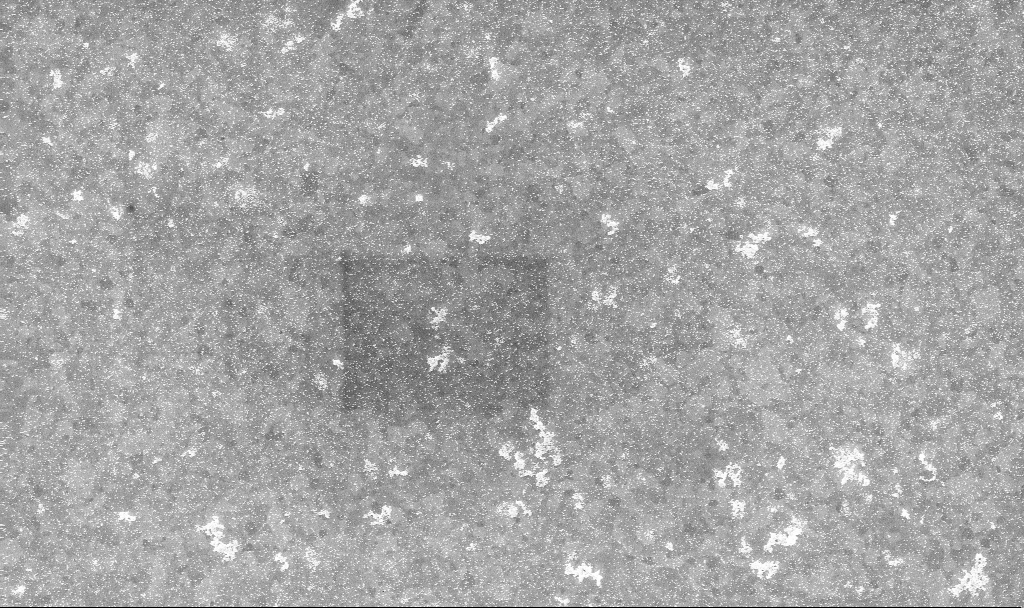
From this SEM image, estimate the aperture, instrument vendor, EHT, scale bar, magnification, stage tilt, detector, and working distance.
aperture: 30 µm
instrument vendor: Zeiss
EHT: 10 kV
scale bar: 1000 nm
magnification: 20 K X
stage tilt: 0°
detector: InLens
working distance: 3.3 mm